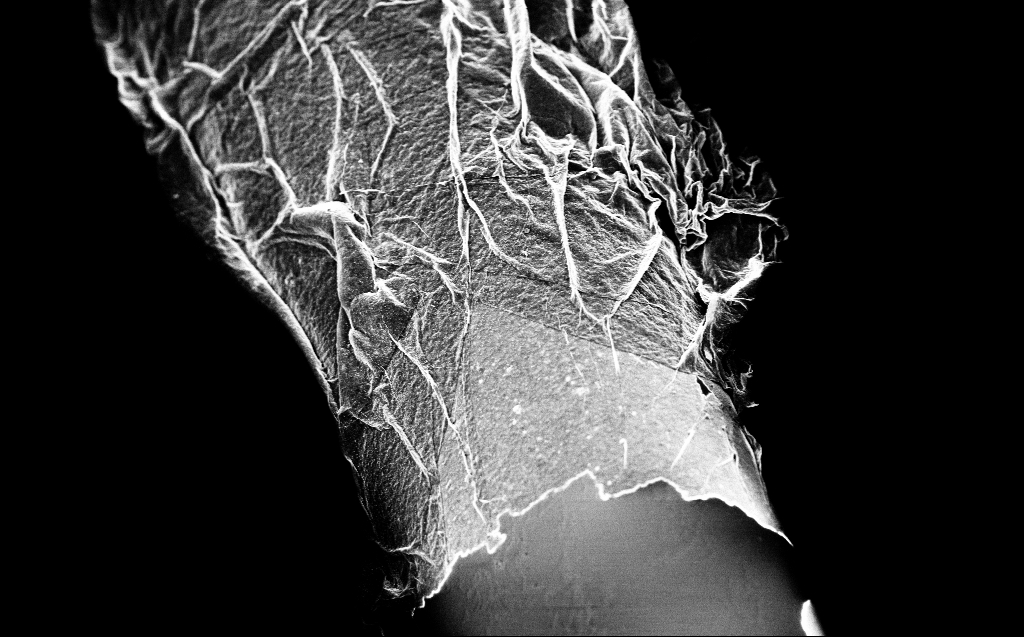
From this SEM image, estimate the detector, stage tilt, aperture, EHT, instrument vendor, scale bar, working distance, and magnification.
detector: InLens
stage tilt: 45°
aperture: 30 µm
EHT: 2 kV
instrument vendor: Zeiss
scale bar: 2000 nm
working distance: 3 mm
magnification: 10 K X